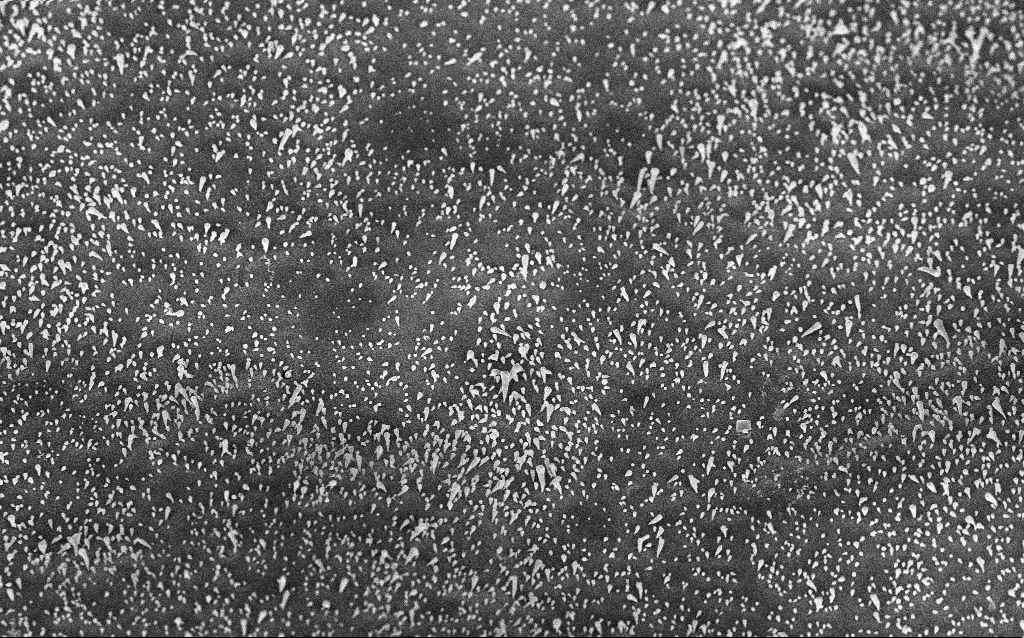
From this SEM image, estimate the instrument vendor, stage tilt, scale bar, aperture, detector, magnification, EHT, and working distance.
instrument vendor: Zeiss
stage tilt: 42.9°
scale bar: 1000 nm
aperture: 30 µm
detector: InLens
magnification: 13.57 K X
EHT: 5 kV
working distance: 6 mm